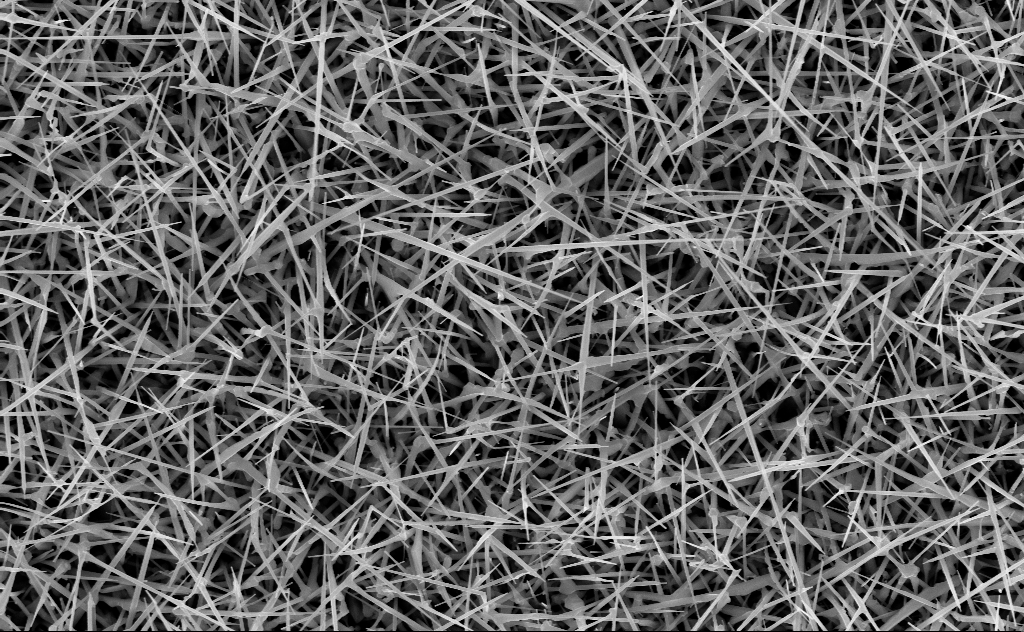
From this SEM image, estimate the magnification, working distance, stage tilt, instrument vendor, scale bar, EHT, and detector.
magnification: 20 K X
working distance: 10 mm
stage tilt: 0°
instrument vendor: Zeiss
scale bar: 2000 nm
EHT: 10 kV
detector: InLens